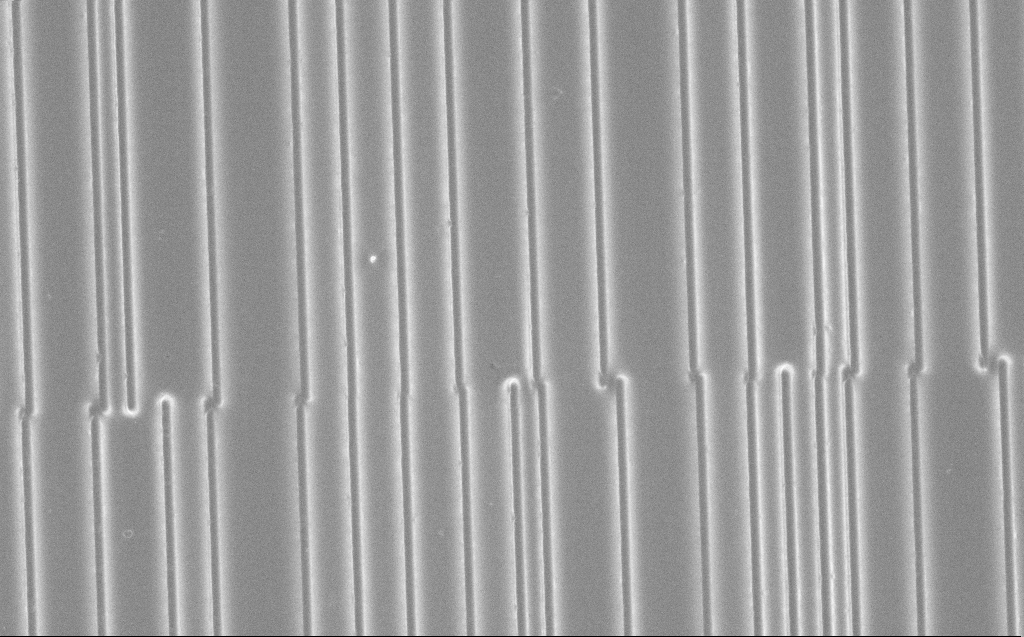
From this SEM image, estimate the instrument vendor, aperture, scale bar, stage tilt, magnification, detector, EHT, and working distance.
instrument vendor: Zeiss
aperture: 30 µm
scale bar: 10000 nm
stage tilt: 0°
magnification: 2.14 K X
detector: InLens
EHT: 5 kV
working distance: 9 mm